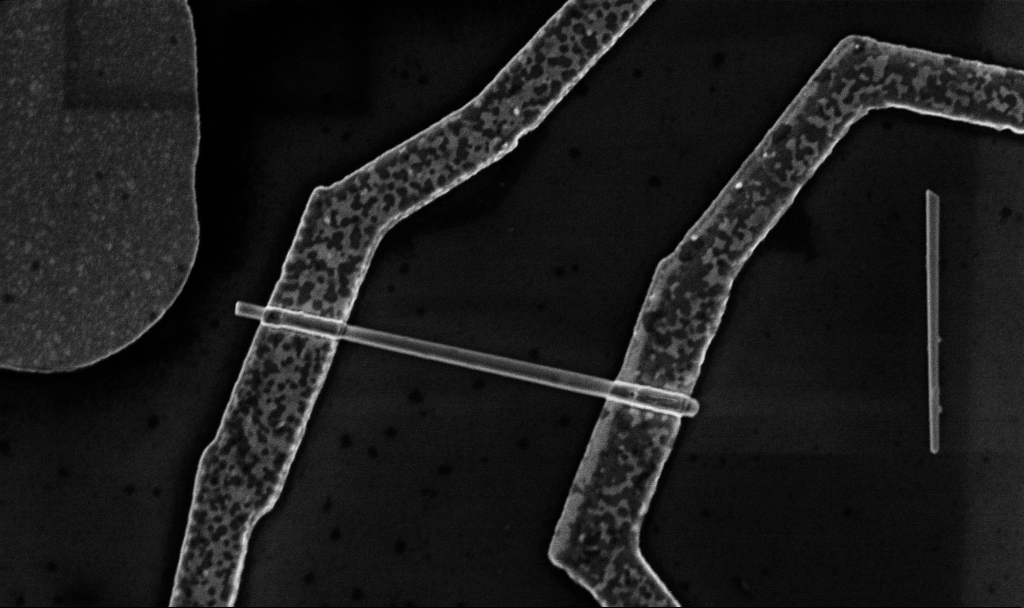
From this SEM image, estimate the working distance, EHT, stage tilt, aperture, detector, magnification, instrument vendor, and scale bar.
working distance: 8.7 mm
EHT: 5 kV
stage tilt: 0°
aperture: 30 µm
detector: InLens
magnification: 30 K X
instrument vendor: Zeiss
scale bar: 1000 nm